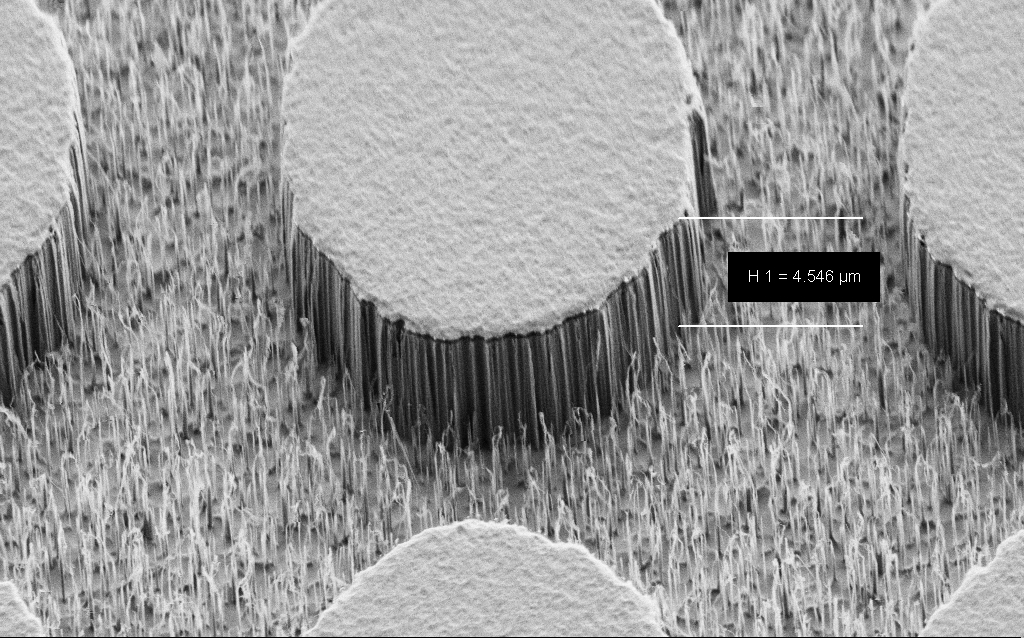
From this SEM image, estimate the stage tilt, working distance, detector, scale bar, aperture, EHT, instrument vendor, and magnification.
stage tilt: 45°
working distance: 7 mm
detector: SE2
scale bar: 2000 nm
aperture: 30 µm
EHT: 2 kV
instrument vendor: Zeiss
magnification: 8.72 K X